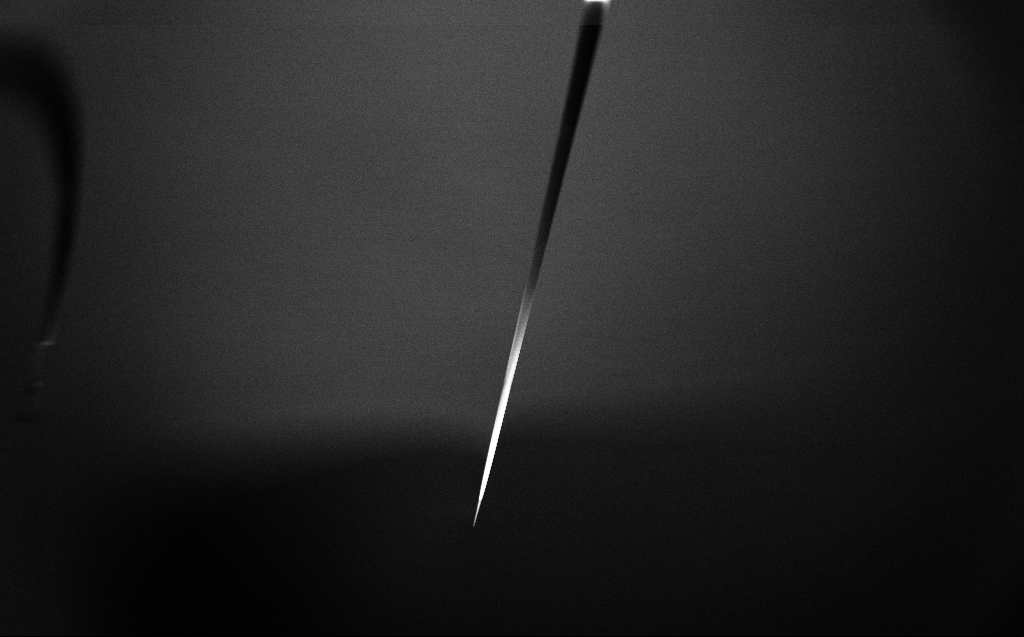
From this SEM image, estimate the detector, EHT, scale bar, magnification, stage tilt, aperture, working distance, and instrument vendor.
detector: InLens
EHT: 2 kV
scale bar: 200000 nm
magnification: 0.1 K X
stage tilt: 45°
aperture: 30 µm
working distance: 4 mm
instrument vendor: Zeiss